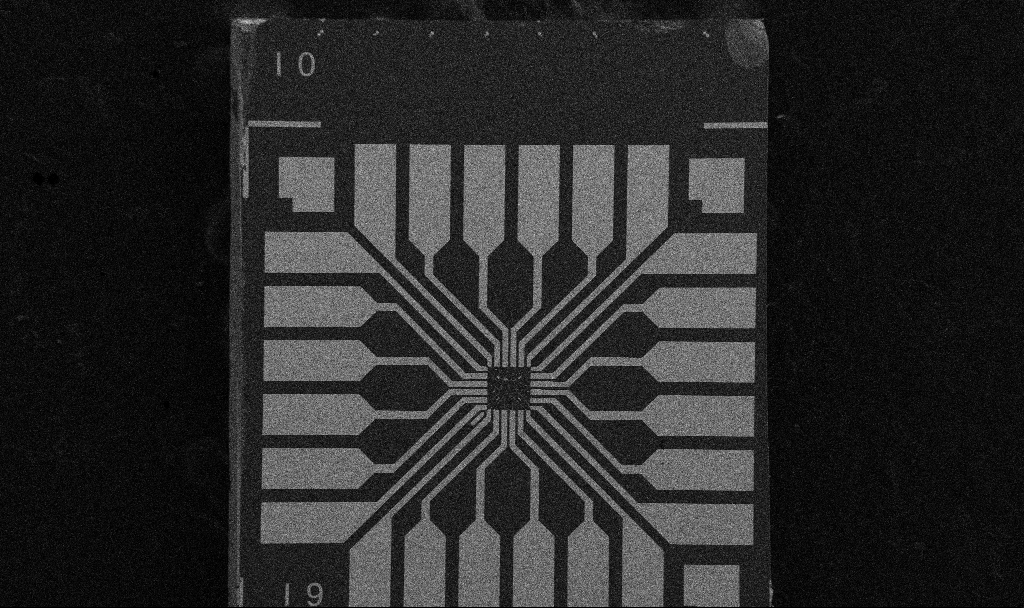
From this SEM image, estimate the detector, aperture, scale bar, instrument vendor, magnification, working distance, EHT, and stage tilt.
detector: SE2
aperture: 30 µm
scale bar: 200000 nm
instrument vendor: Zeiss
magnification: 0.1 K X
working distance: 10.5 mm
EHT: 5 kV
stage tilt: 0°